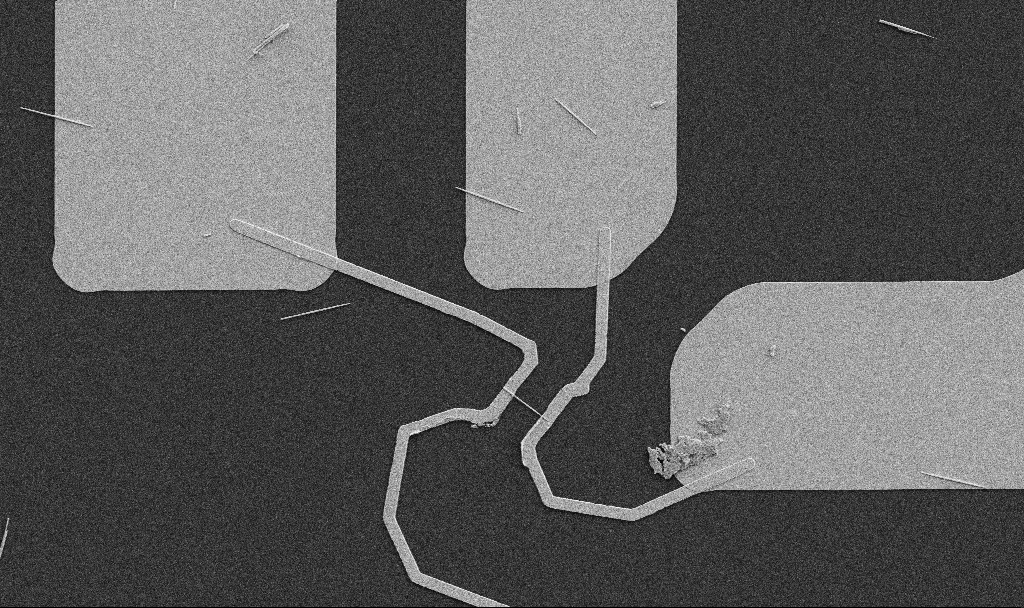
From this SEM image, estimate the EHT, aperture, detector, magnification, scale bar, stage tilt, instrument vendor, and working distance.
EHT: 5 kV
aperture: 30 µm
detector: SE2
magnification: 5 K X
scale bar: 10000 nm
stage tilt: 0°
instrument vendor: Zeiss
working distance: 10.7 mm